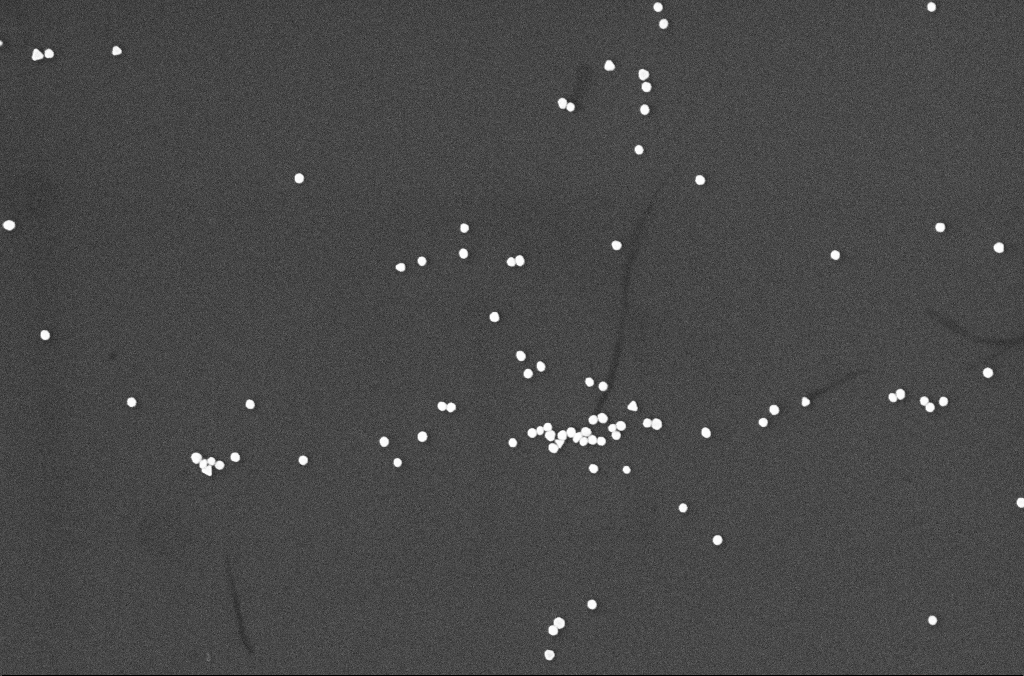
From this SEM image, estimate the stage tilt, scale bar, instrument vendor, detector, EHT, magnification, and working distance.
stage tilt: -0°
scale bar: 1000 nm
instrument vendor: Zeiss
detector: InLens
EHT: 10 kV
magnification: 54.7 K X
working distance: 4.9 mm